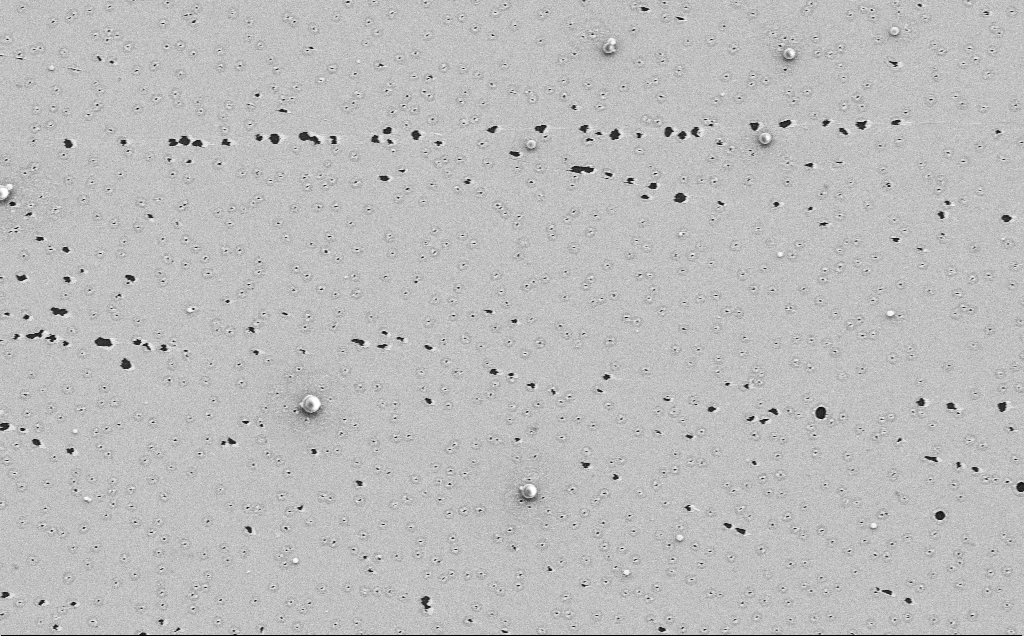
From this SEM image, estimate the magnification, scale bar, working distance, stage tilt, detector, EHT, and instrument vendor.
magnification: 4.2 K X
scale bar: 10000 nm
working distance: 12 mm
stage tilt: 0°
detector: SE2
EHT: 5 kV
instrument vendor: Zeiss